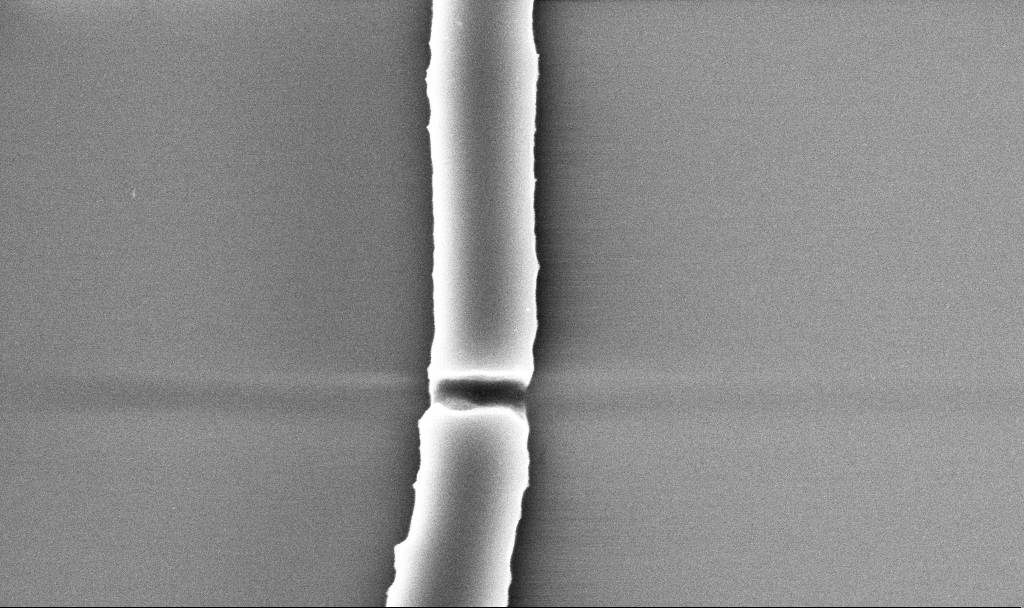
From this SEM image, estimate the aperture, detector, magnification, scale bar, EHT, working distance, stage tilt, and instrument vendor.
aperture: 30 µm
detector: InLens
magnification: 76.86 K X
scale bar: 200 nm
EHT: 5 kV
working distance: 5.2 mm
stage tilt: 0°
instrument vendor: Zeiss